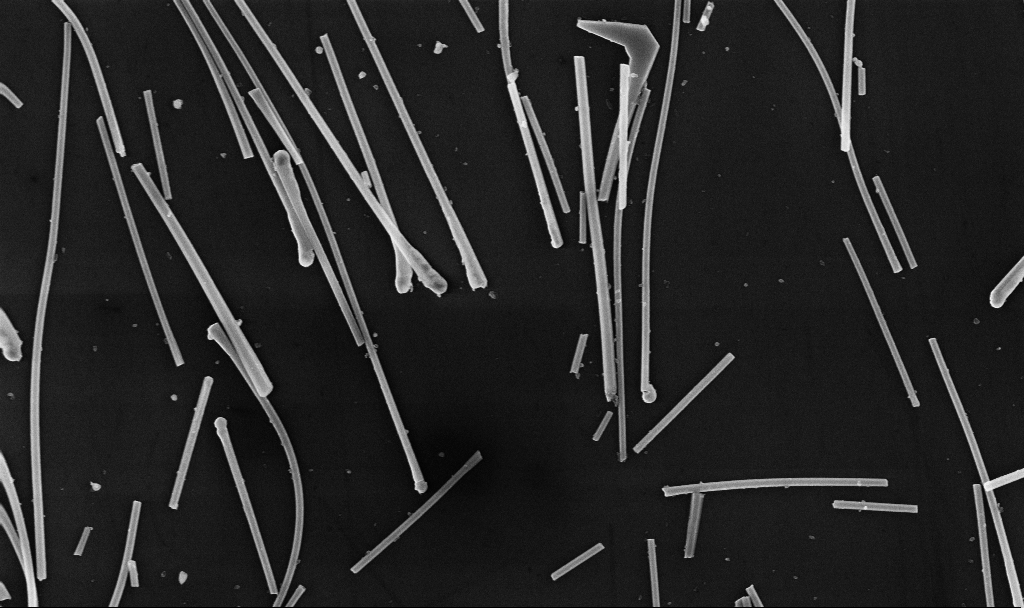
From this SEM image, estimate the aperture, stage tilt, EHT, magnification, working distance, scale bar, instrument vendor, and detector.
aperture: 30 µm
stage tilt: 0°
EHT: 10 kV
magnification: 27.19 K X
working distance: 6.7 mm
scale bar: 1000 nm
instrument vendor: Zeiss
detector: InLens